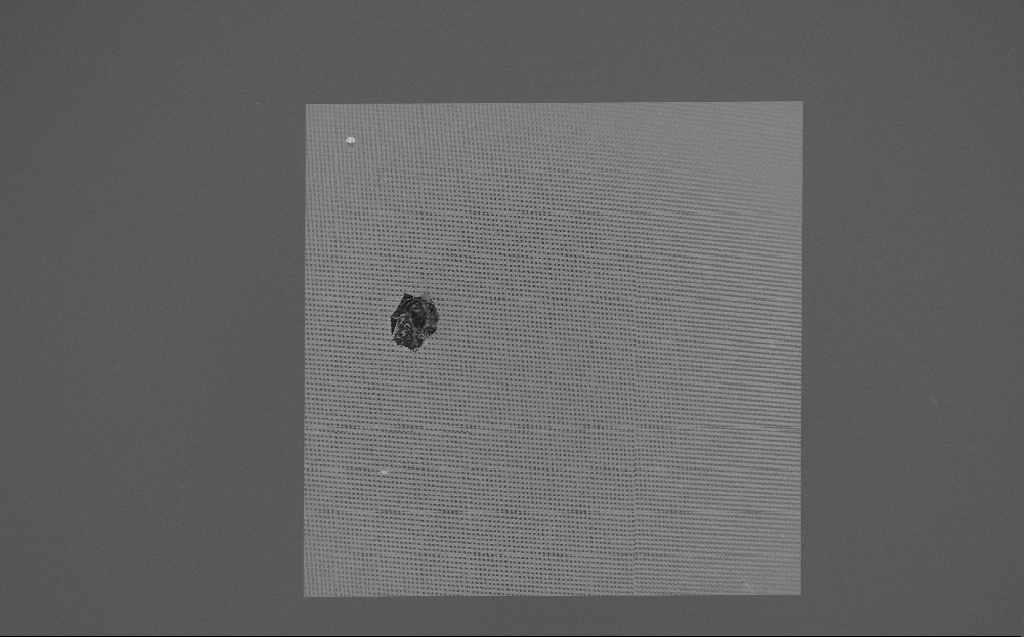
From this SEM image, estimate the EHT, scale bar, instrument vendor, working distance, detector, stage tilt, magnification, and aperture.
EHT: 5 kV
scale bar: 100000 nm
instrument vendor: Zeiss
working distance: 7 mm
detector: InLens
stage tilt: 0°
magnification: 0.244 K X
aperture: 30 µm